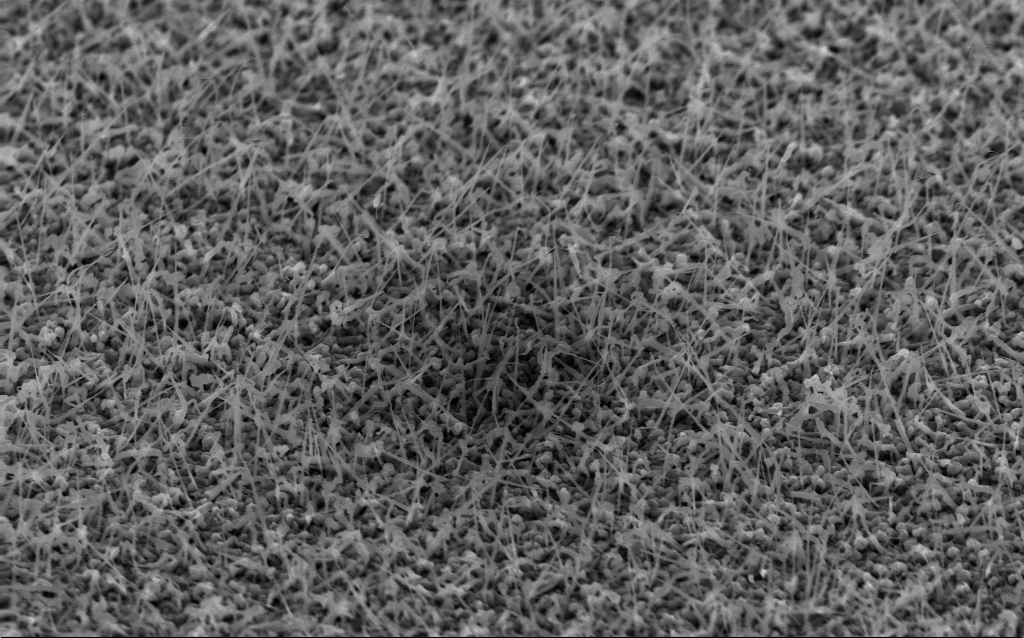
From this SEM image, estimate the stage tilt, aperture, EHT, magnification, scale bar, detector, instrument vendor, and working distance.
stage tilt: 53°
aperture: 30 µm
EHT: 10 kV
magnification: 24.15 K X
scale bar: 1000 nm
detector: InLens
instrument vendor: Zeiss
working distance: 3 mm